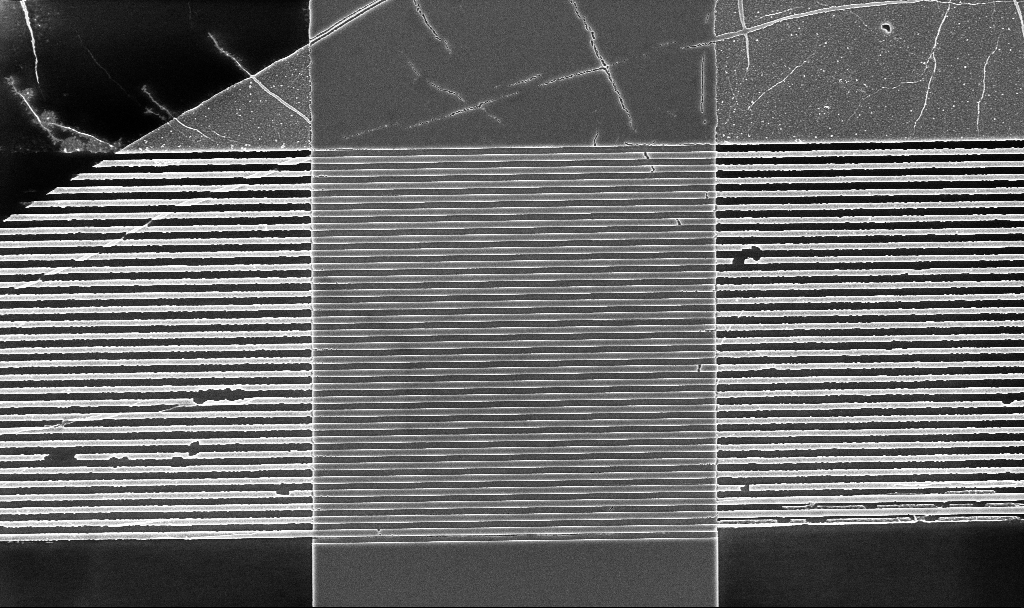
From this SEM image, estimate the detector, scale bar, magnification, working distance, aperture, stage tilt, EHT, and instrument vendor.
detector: InLens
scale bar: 2000 nm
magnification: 7.54 K X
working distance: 5.2 mm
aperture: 30 µm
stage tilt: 0°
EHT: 5 kV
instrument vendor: Zeiss